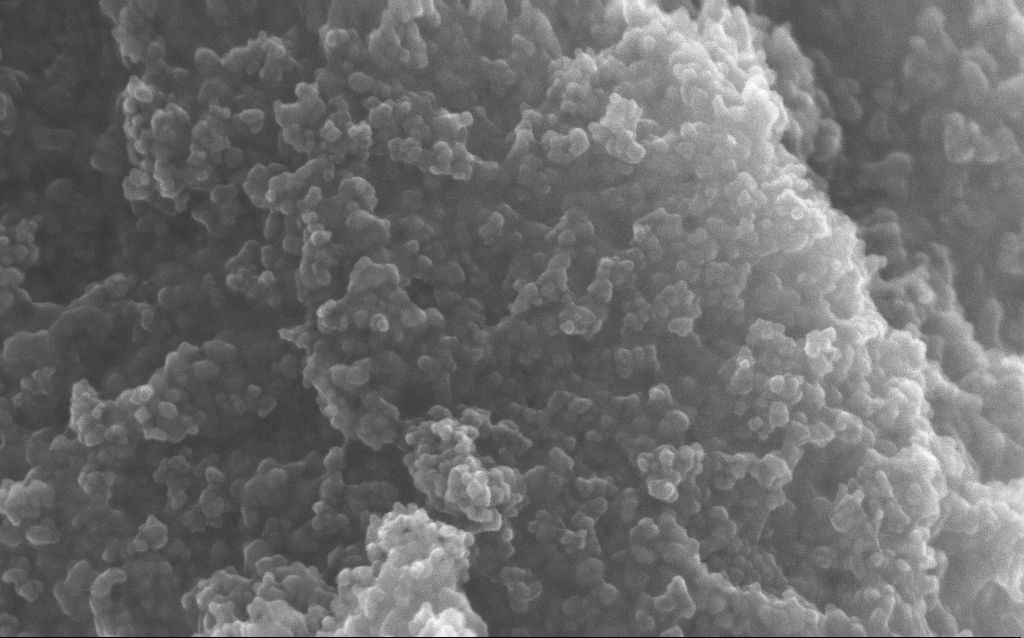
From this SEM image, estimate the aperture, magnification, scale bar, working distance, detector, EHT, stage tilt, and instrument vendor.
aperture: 30 µm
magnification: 211.33 K X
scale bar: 100 nm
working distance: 2.7 mm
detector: InLens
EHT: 10 kV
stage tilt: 0°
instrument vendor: Zeiss